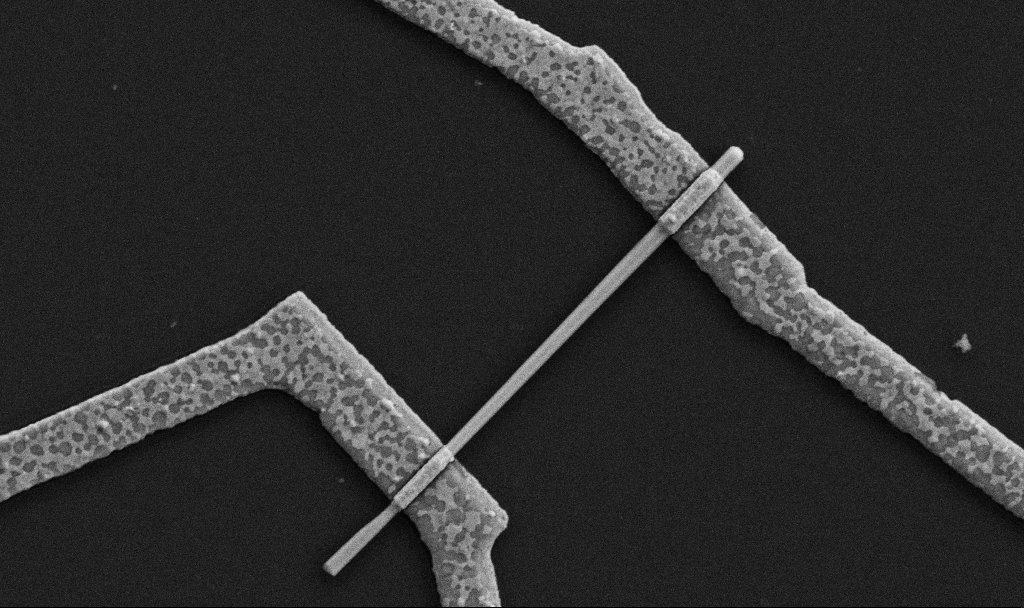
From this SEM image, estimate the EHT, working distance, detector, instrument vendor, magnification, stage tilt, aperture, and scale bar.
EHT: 5 kV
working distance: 8.7 mm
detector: SE2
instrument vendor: Zeiss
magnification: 30 K X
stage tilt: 0°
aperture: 30 µm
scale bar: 1000 nm